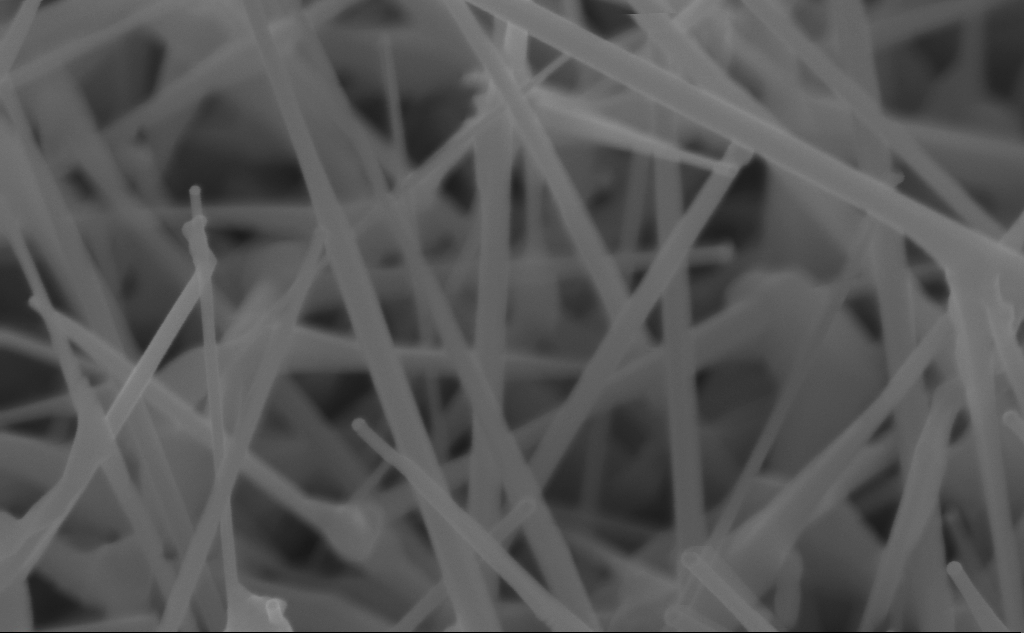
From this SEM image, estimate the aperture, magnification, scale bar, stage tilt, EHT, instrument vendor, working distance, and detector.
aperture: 30 µm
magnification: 150 K X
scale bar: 200 nm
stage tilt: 45°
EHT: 10 kV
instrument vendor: Zeiss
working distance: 4 mm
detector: InLens